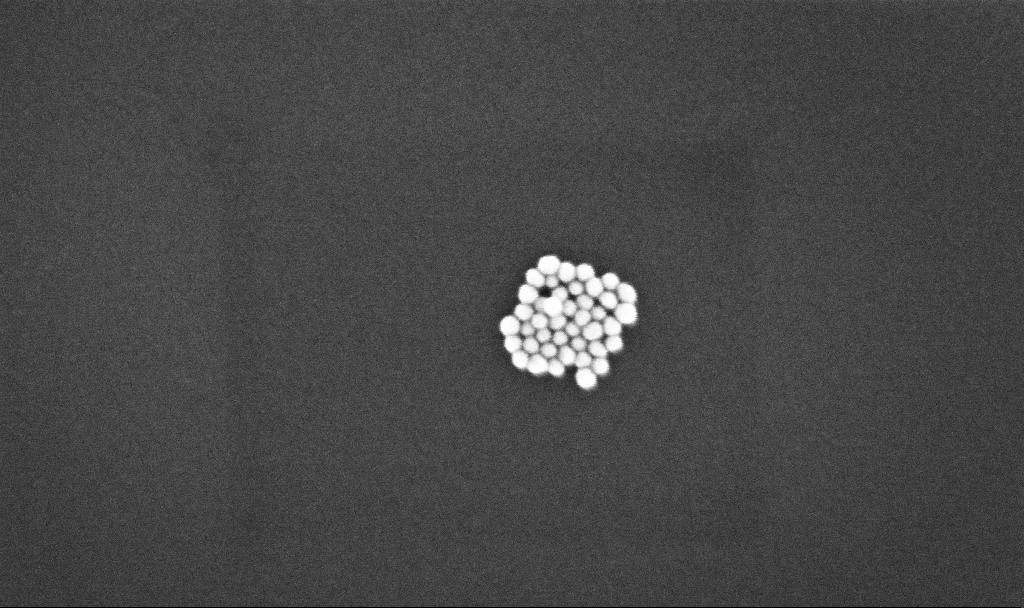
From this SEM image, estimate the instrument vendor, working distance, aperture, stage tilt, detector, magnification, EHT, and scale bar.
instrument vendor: Zeiss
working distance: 3.3 mm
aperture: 30 µm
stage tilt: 0°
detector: InLens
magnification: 317.3 K X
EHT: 10 kV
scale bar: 200 nm